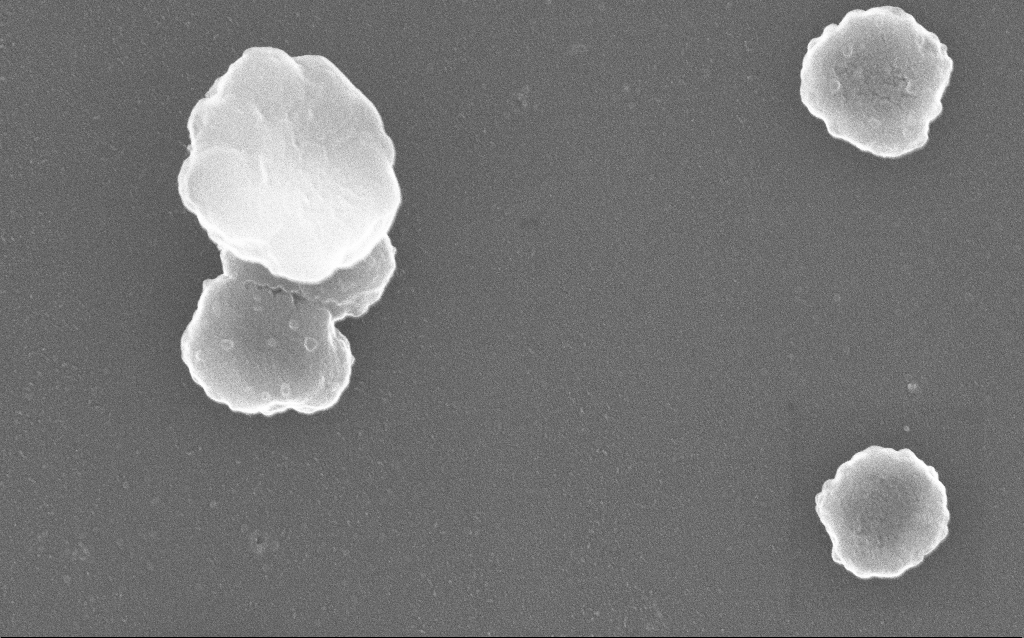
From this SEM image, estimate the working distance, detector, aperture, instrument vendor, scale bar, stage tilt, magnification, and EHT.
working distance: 1.4 mm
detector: InLens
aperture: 30 µm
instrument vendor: Zeiss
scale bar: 200 nm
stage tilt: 0°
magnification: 100 K X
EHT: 20 kV